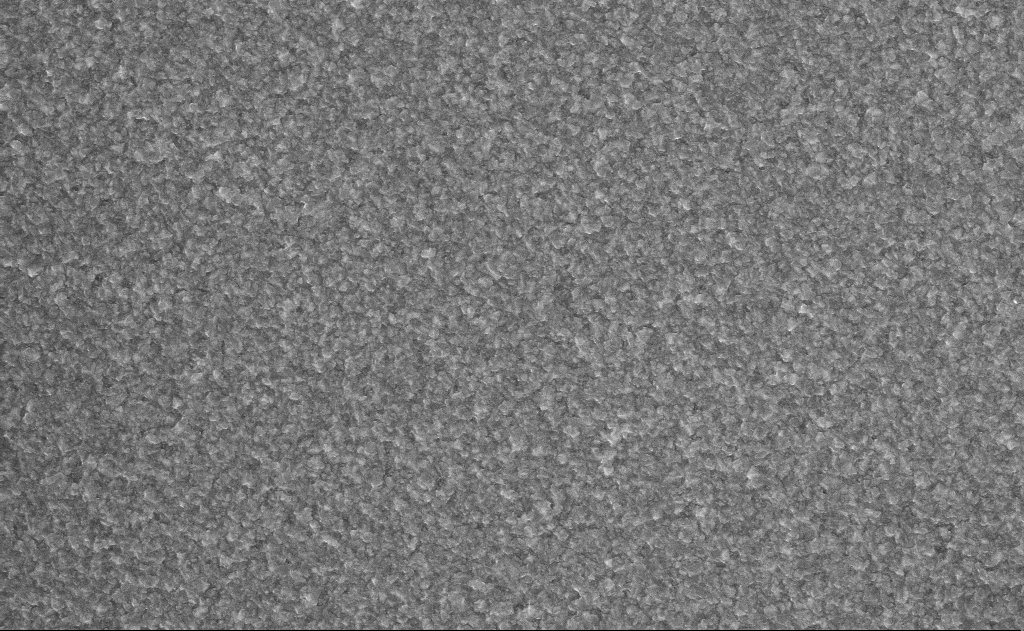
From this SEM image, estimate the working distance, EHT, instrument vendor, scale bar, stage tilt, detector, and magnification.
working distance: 16 mm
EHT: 10 kV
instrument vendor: Zeiss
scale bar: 1000 nm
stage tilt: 0°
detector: InLens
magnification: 20 K X